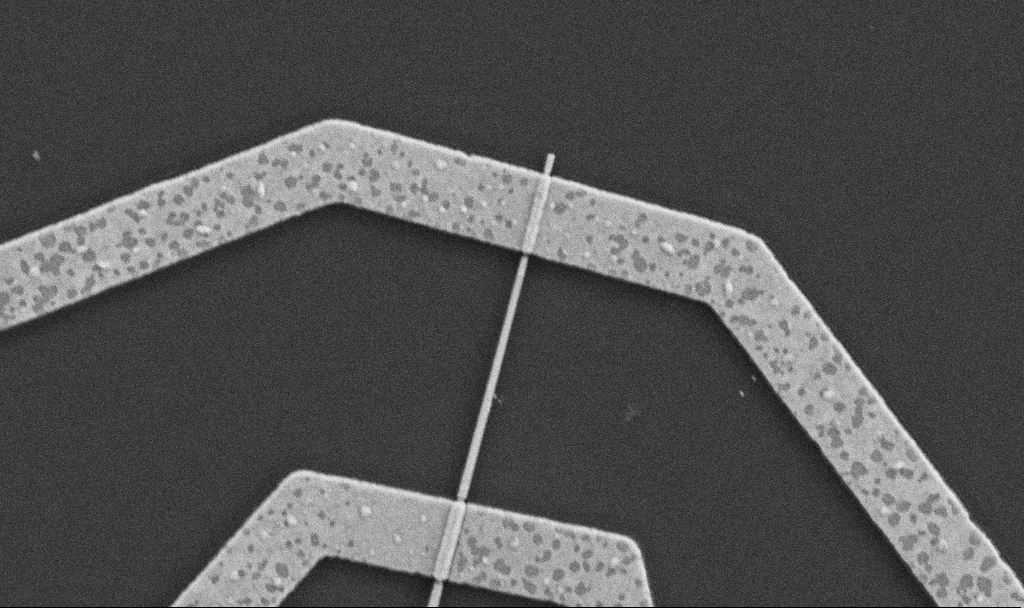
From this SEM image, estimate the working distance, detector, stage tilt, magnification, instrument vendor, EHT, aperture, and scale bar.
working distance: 8.7 mm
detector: SE2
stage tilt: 0°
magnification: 30 K X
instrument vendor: Zeiss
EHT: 5 kV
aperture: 30 µm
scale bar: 1000 nm